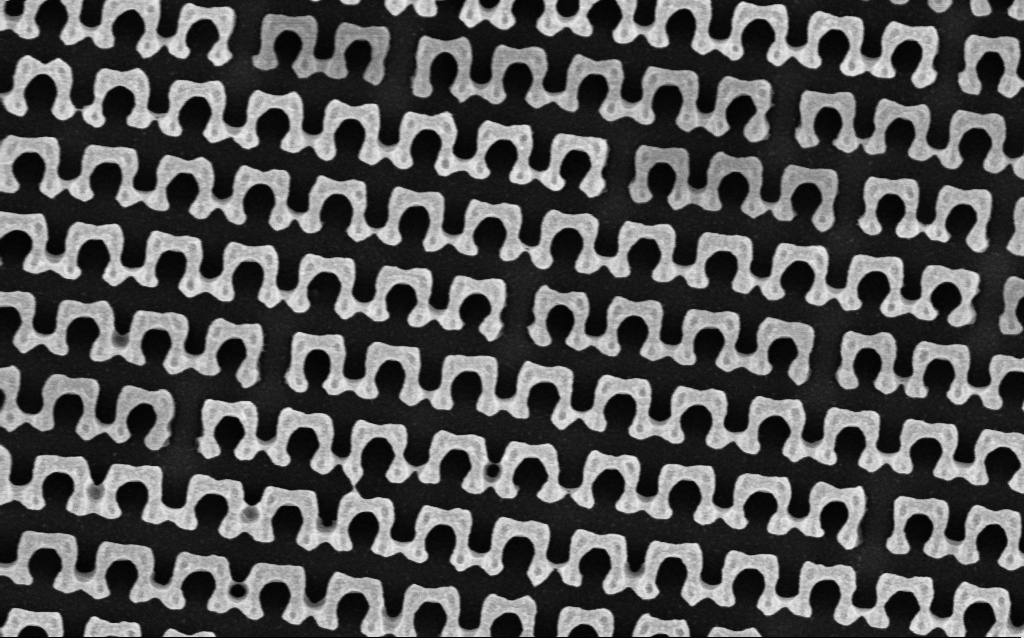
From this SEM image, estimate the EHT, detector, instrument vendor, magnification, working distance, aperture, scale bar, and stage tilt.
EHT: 3 kV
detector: InLens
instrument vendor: Zeiss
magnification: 62.08 K X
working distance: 4.6 mm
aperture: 30 µm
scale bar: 1000 nm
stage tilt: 0°